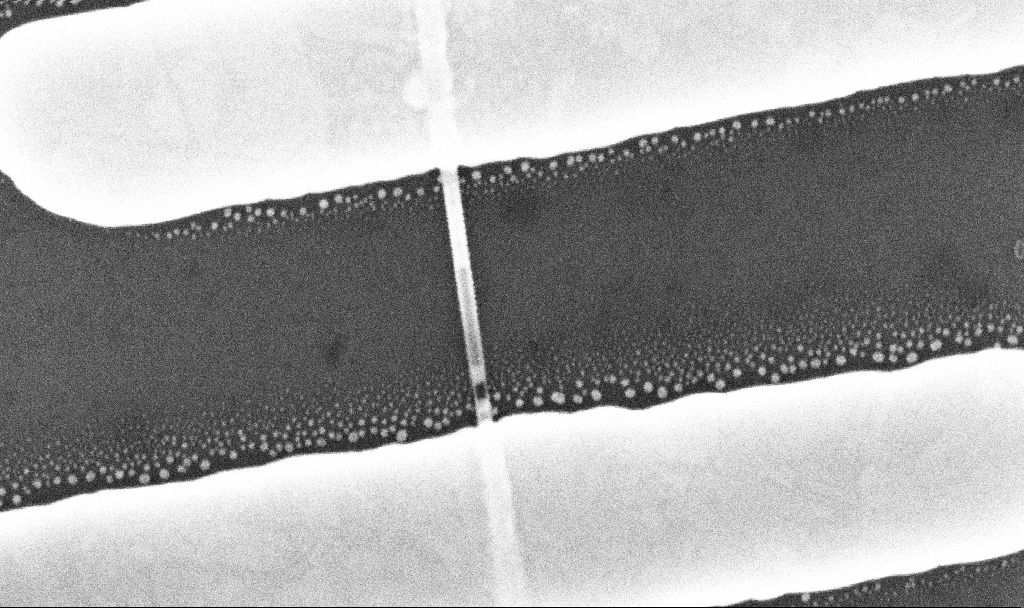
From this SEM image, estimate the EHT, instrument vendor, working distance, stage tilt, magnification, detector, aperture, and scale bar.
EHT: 10 kV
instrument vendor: Zeiss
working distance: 7 mm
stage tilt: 0°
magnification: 197.82 K X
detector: InLens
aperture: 30 µm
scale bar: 200 nm